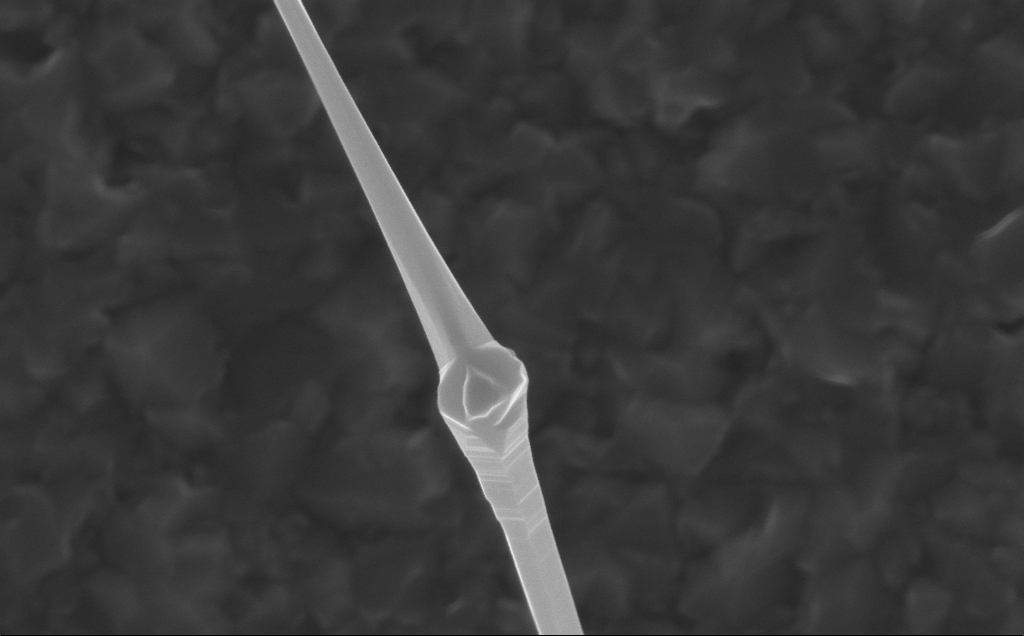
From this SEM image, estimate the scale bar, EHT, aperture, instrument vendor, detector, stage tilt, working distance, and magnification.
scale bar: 200 nm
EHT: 10 kV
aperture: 30 µm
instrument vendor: Zeiss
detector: InLens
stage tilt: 0°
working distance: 5 mm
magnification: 80 K X